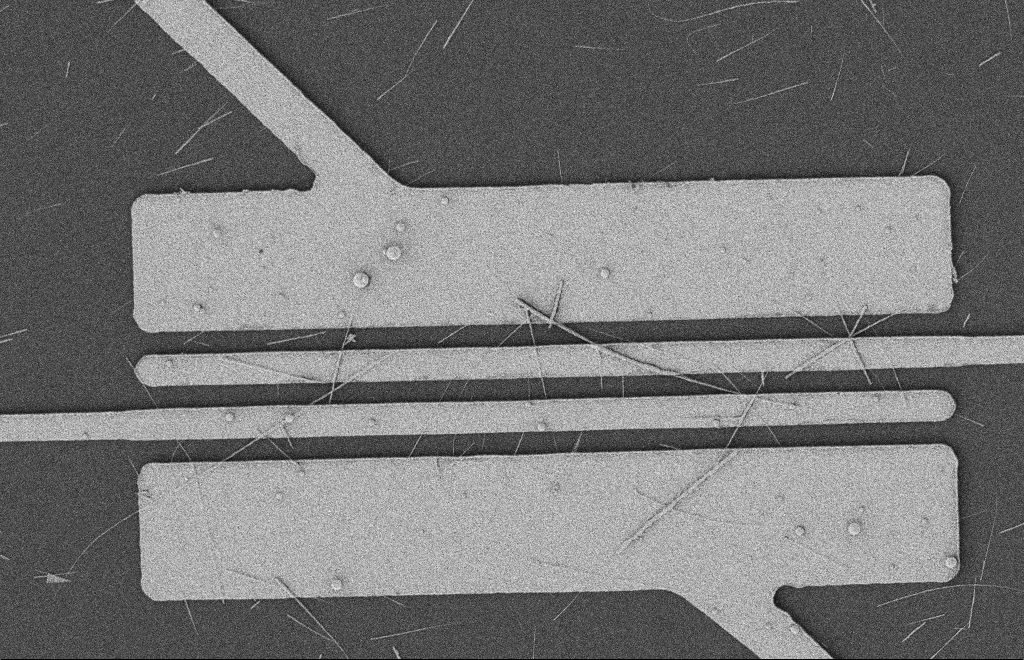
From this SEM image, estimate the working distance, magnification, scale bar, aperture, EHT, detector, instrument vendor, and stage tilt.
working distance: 12 mm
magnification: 4.9 K X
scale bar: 2000 nm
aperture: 20 µm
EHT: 2 kV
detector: SE2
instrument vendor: Zeiss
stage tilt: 0°